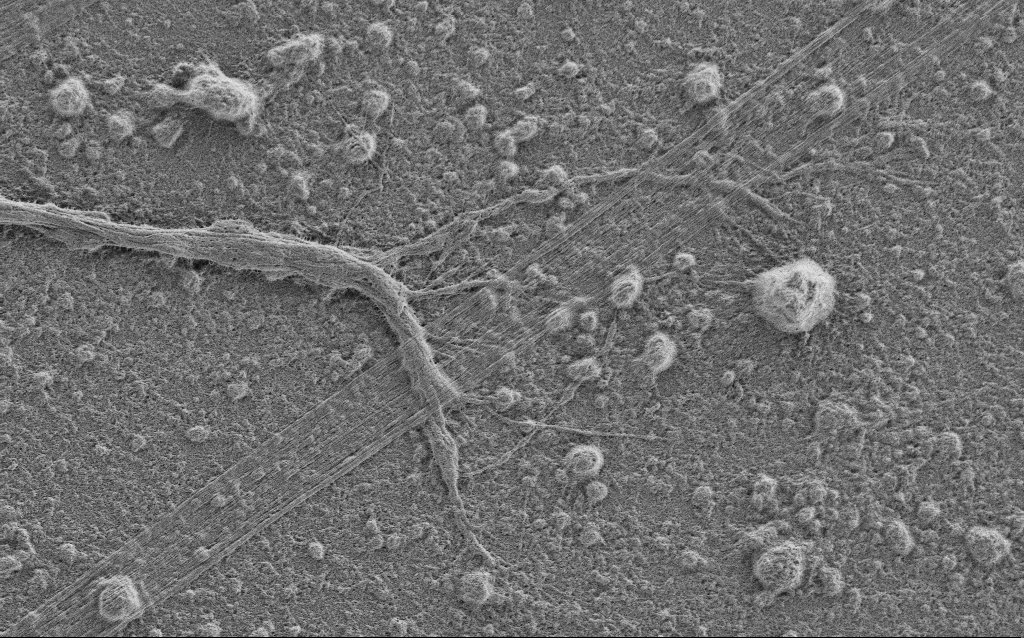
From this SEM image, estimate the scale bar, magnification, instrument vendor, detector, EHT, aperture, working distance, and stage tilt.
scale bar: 10000 nm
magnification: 5 K X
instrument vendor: Zeiss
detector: SE2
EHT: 0.9 kV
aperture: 30 µm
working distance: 4 mm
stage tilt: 0°